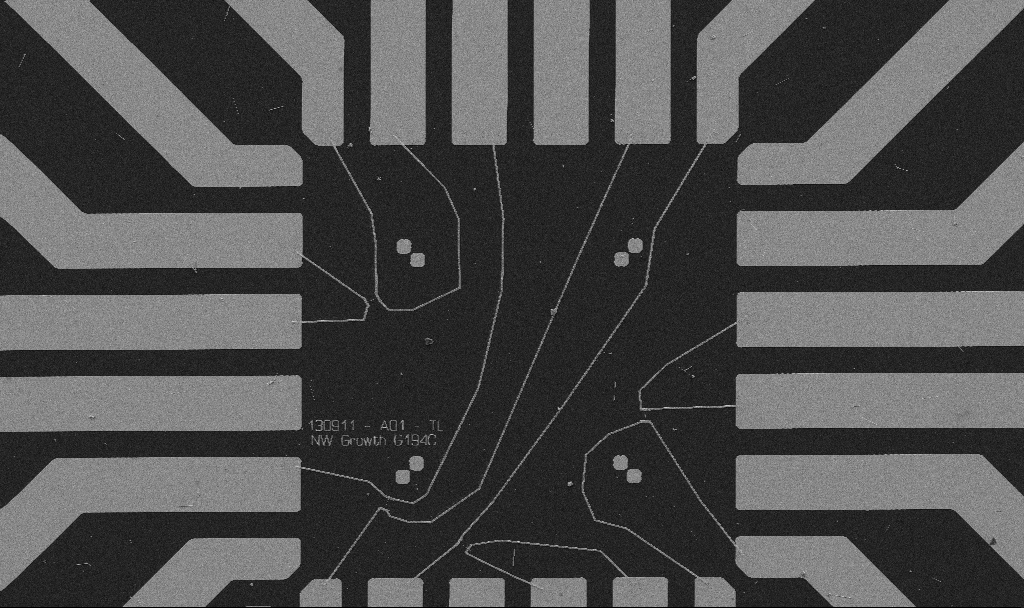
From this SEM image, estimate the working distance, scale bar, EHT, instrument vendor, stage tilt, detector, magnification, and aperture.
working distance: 10.7 mm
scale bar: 20000 nm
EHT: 5 kV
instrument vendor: Zeiss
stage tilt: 0°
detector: SE2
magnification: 1 K X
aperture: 30 µm